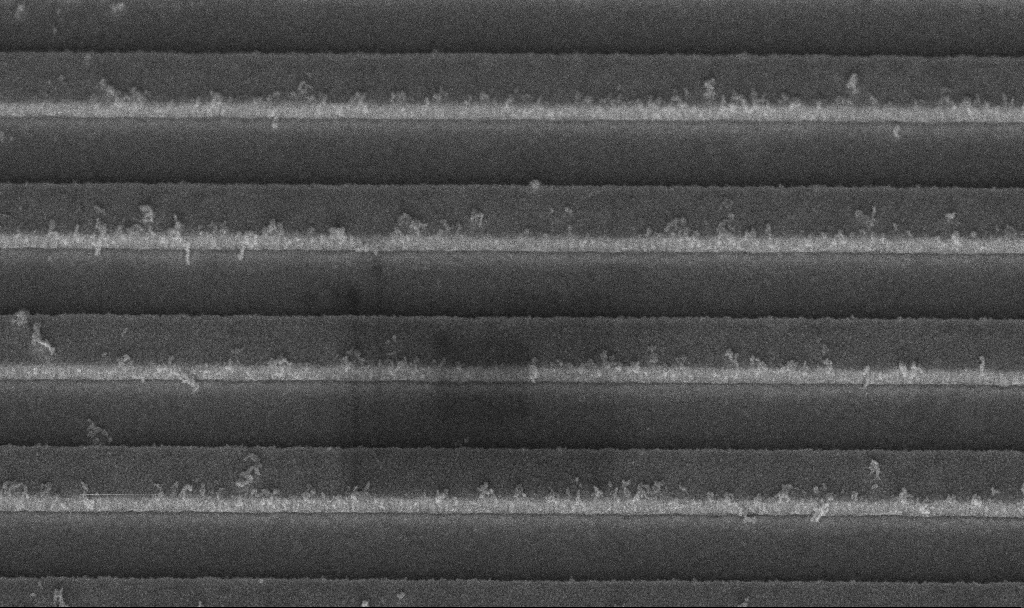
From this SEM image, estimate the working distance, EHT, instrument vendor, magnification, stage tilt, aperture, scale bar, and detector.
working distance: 8 mm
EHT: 5 kV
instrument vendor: Zeiss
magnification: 62.42 K X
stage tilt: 45°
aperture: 30 µm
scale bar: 1000 nm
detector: InLens